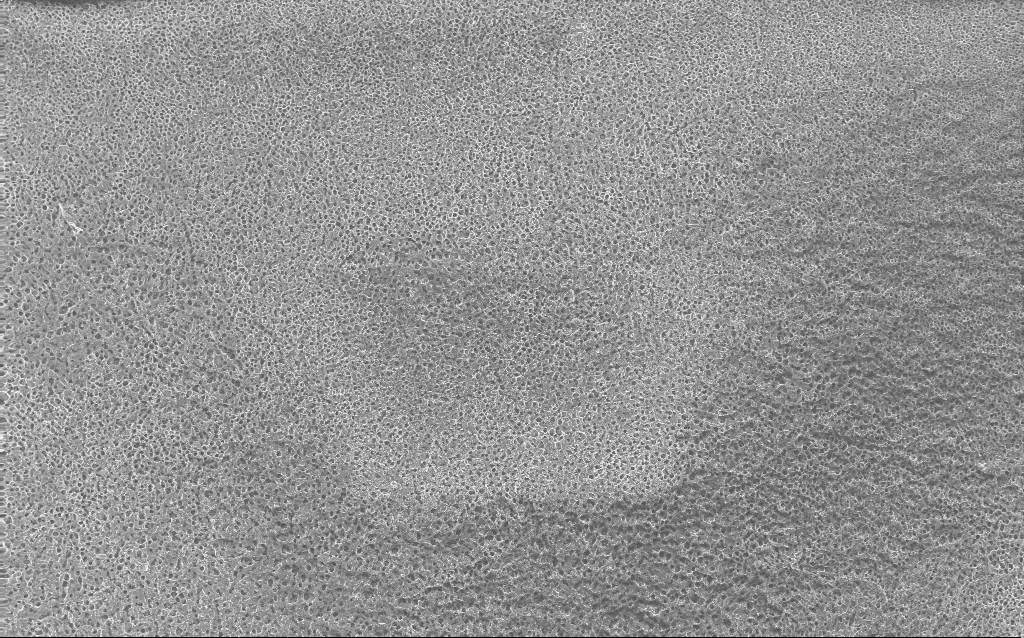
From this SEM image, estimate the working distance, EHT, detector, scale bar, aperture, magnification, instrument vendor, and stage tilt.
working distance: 2.4 mm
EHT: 10 kV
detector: InLens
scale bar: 100000 nm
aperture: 30 µm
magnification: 0.235 K X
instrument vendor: Zeiss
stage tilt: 0°